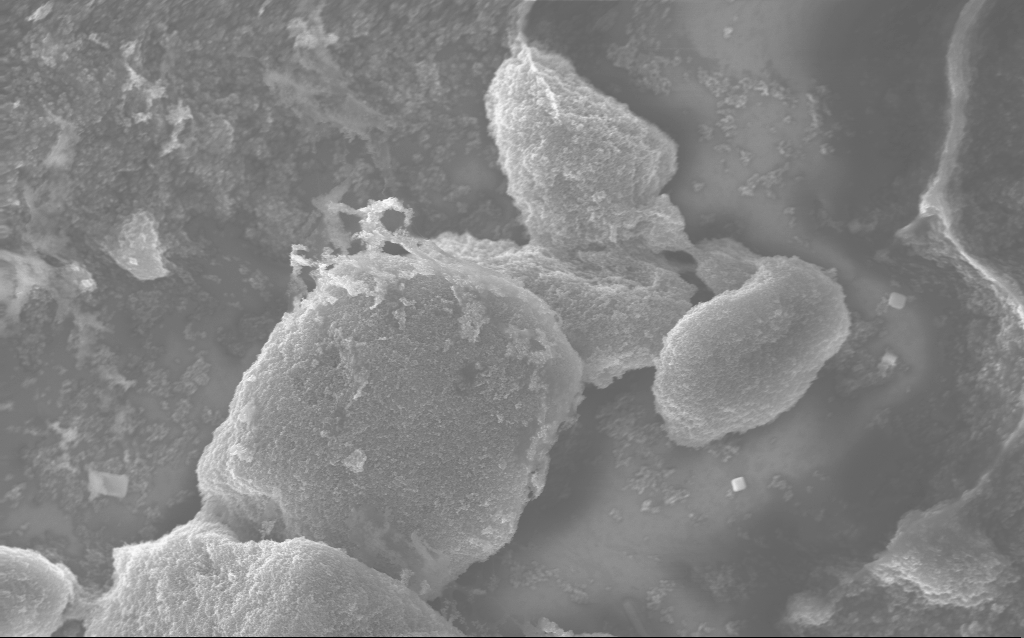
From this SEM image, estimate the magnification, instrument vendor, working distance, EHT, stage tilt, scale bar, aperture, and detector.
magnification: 20.87 K X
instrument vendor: Zeiss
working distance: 2.8 mm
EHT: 10 kV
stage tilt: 0°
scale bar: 1000 nm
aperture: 30 µm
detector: InLens